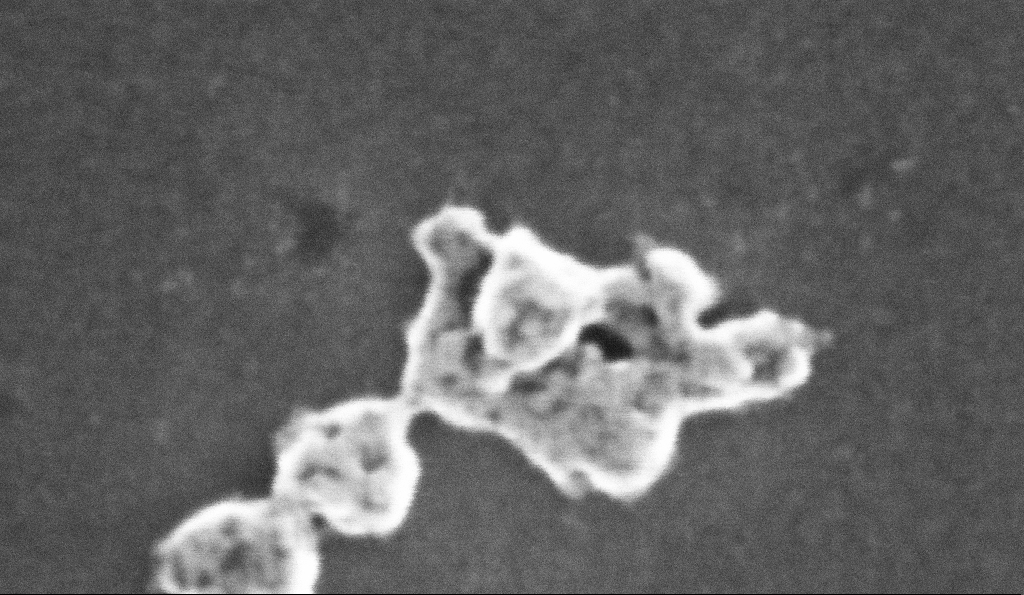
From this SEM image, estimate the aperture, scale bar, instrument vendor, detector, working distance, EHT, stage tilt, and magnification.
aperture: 30 µm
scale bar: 20 nm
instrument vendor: Zeiss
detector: InLens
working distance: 5.3 mm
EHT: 10 kV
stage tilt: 0°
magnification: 887.31 K X